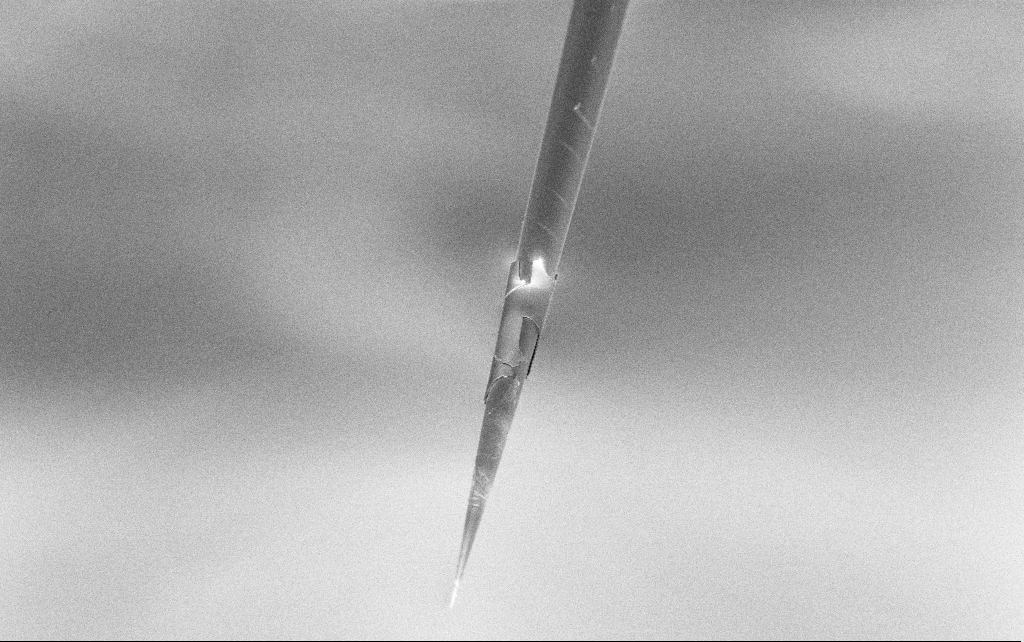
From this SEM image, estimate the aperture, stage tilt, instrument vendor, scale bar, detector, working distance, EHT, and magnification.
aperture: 30 µm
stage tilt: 45°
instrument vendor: Zeiss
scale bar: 20000 nm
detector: InLens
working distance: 7.4 mm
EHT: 3 kV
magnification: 1 K X